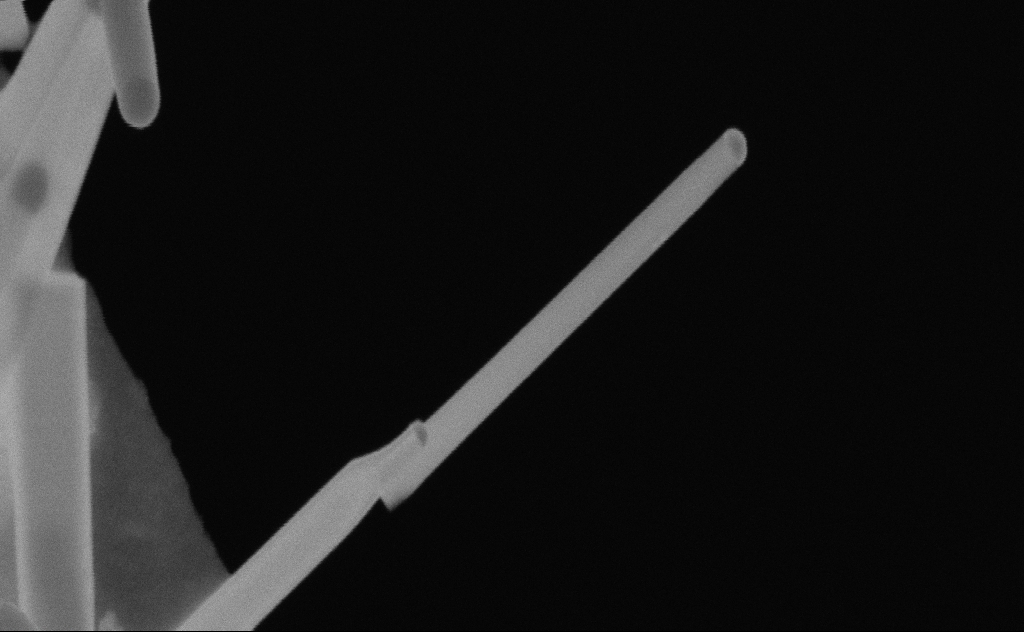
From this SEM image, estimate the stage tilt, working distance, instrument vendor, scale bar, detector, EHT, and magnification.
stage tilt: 0°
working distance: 9 mm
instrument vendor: Zeiss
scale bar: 200 nm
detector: SE2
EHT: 20 kV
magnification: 246.52 K X